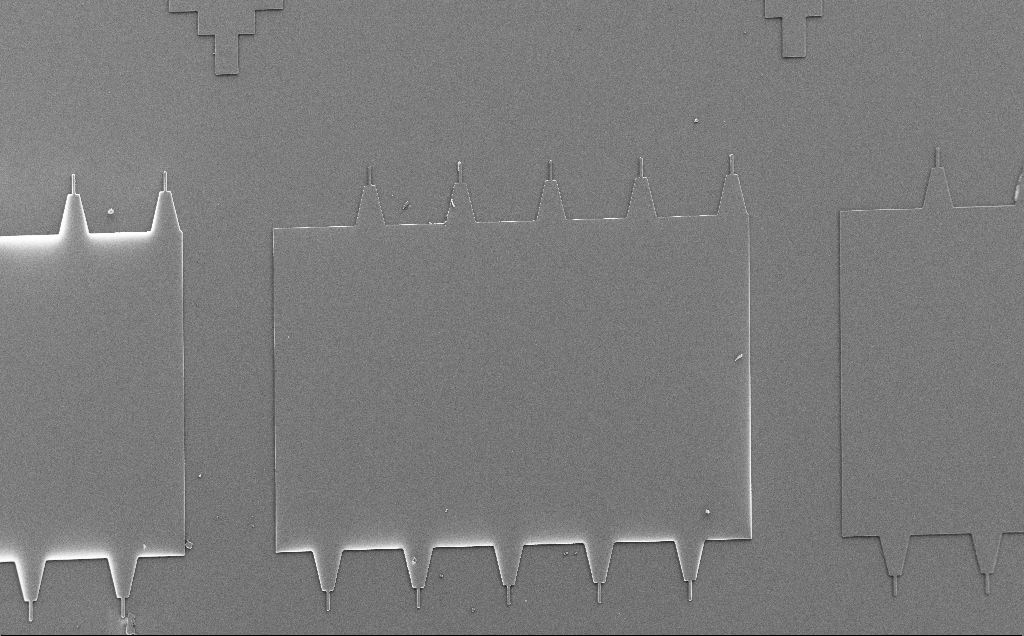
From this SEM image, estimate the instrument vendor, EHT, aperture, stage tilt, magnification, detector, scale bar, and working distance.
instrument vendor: Zeiss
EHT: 5 kV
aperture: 30 µm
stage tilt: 0°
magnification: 0.21 K X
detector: SE2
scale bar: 100000 nm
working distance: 7 mm